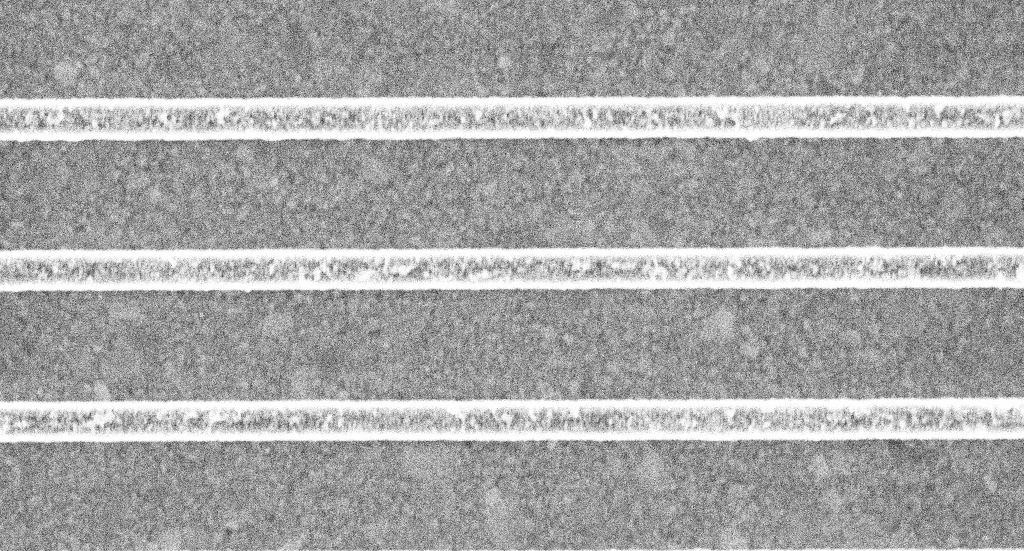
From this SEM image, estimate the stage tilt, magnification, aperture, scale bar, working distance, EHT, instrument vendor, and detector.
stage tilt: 0°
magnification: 79.85 K X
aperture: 30 µm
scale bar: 200 nm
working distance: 3.1 mm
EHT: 5 kV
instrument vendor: Zeiss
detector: InLens